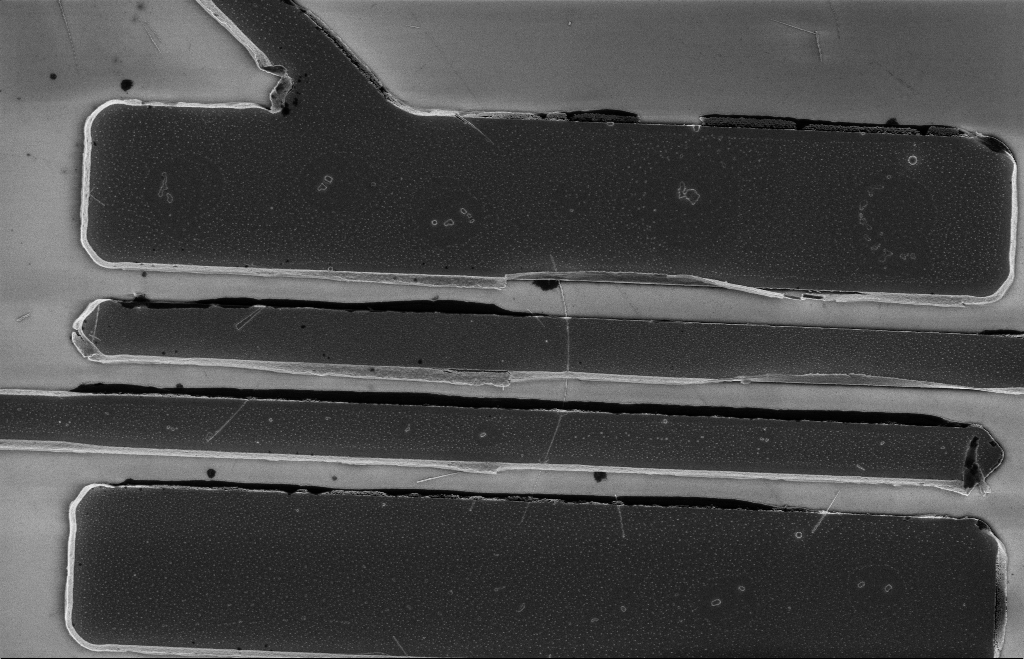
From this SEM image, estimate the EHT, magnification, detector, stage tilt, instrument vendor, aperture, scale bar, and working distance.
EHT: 5 kV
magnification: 5.65 K X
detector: InLens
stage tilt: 0°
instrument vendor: Zeiss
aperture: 20 µm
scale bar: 2000 nm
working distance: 12 mm